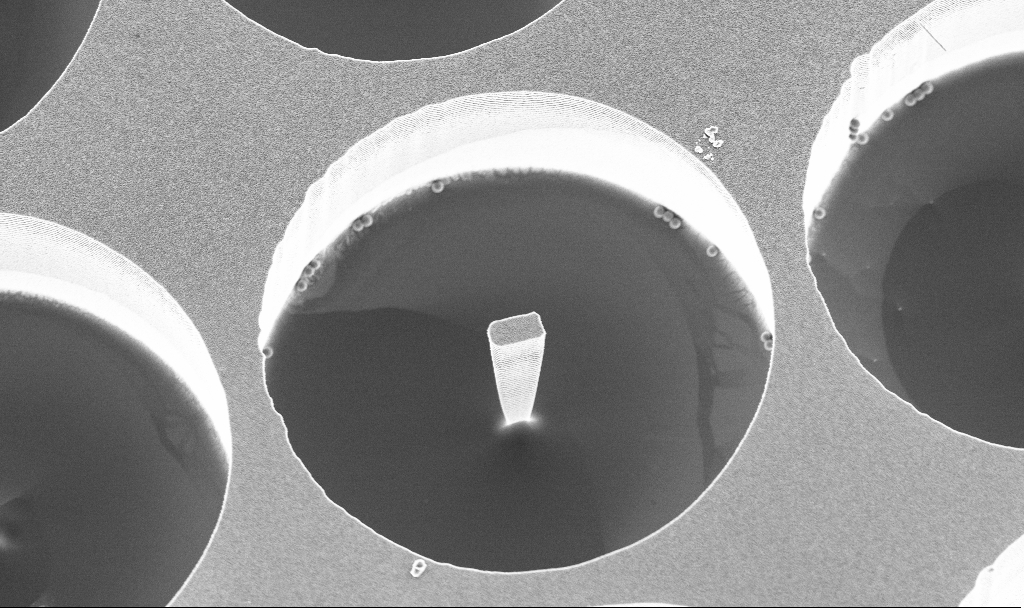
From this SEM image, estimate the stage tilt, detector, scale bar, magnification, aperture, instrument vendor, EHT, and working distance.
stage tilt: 20°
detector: InLens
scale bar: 10000 nm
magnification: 6.22 K X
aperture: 30 µm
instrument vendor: Zeiss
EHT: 3 kV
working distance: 3.8 mm